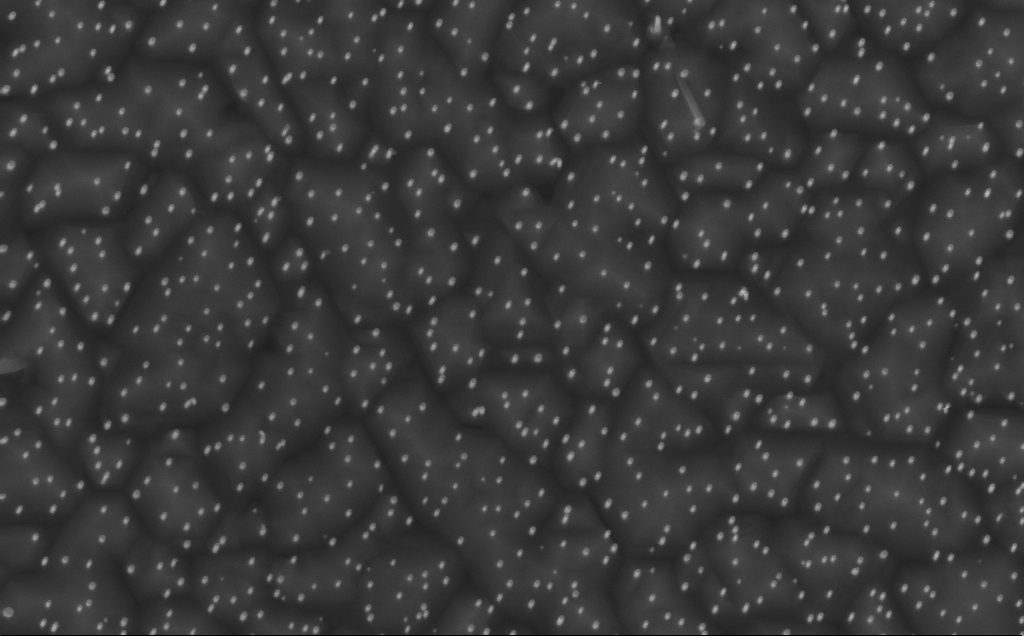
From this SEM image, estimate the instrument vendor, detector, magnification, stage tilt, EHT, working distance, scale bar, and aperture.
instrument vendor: Zeiss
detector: InLens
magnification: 40 K X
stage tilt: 0°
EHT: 10 kV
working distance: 5 mm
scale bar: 1000 nm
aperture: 30 µm